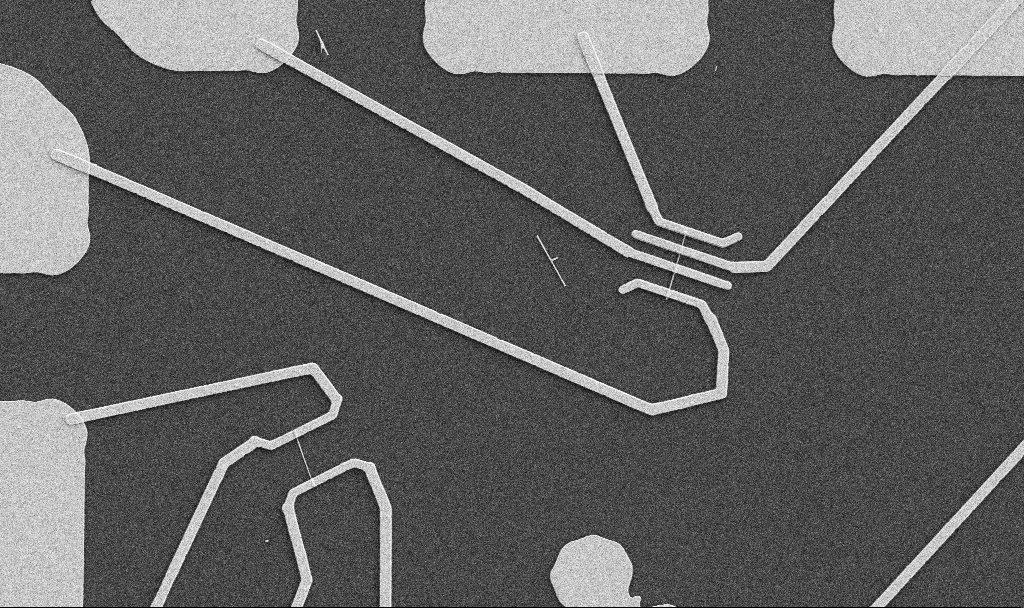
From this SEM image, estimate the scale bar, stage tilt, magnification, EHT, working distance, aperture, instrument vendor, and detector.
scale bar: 10000 nm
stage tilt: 0°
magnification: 5 K X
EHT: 5 kV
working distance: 10.7 mm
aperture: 30 µm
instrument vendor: Zeiss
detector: SE2